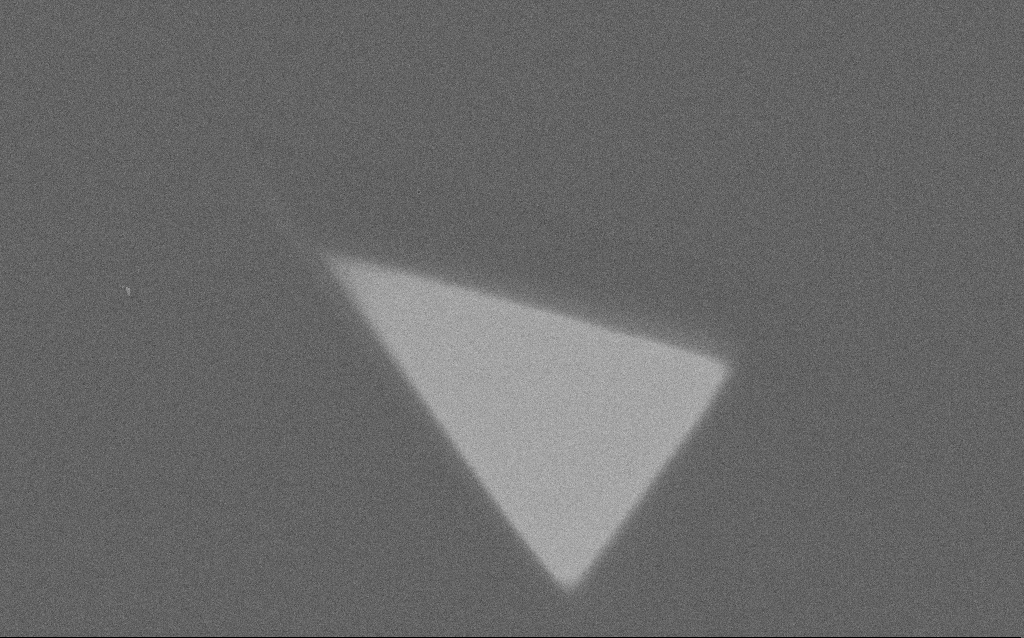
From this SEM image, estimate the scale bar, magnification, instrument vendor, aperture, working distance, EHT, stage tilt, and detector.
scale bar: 10000 nm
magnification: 7.23 K X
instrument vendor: Zeiss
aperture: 30 µm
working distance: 6 mm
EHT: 1.5 kV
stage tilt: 0°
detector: SE2